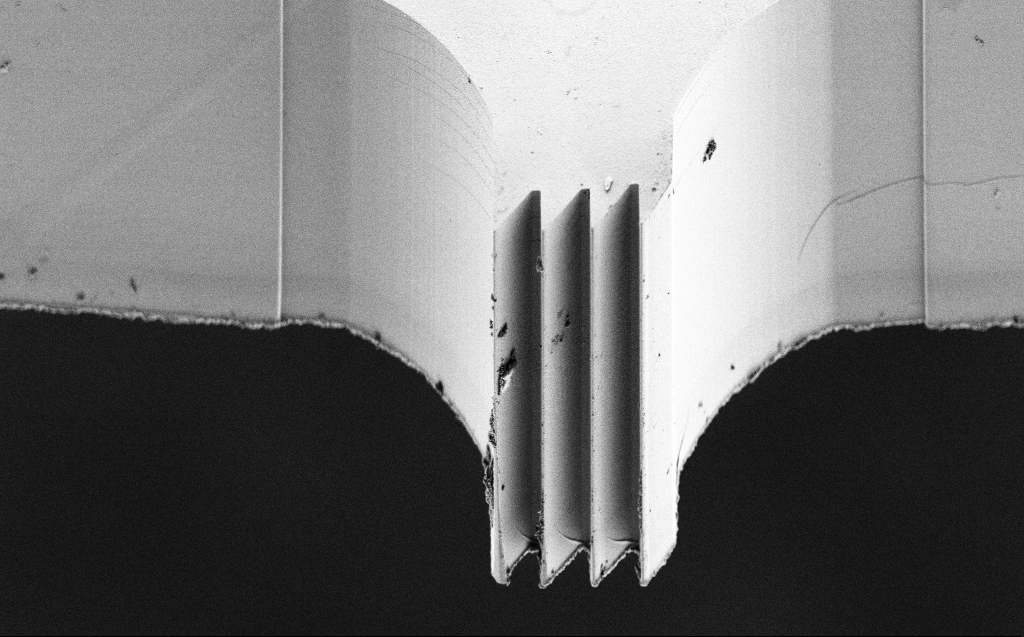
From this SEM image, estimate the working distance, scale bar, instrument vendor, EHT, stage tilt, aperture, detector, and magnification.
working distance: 8 mm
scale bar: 10000 nm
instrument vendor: Zeiss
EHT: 3 kV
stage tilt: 45°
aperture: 30 µm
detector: SE2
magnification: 1.6 K X